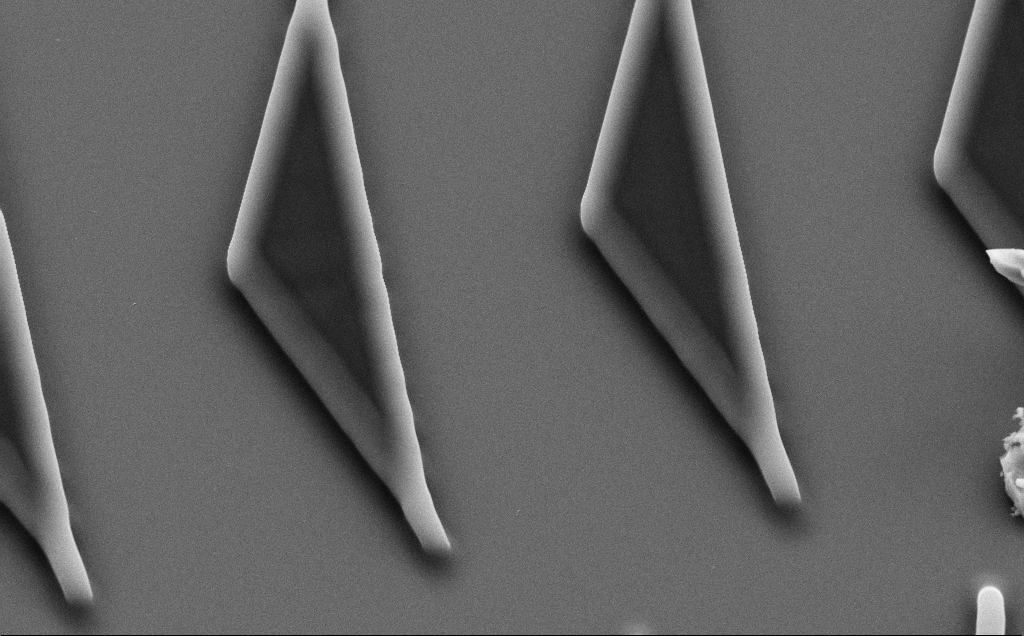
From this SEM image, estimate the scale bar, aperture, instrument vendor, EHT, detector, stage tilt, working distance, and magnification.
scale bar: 2000 nm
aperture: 30 µm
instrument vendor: Zeiss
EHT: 10 kV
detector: SE2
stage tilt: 35°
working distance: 8 mm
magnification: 7.75 K X